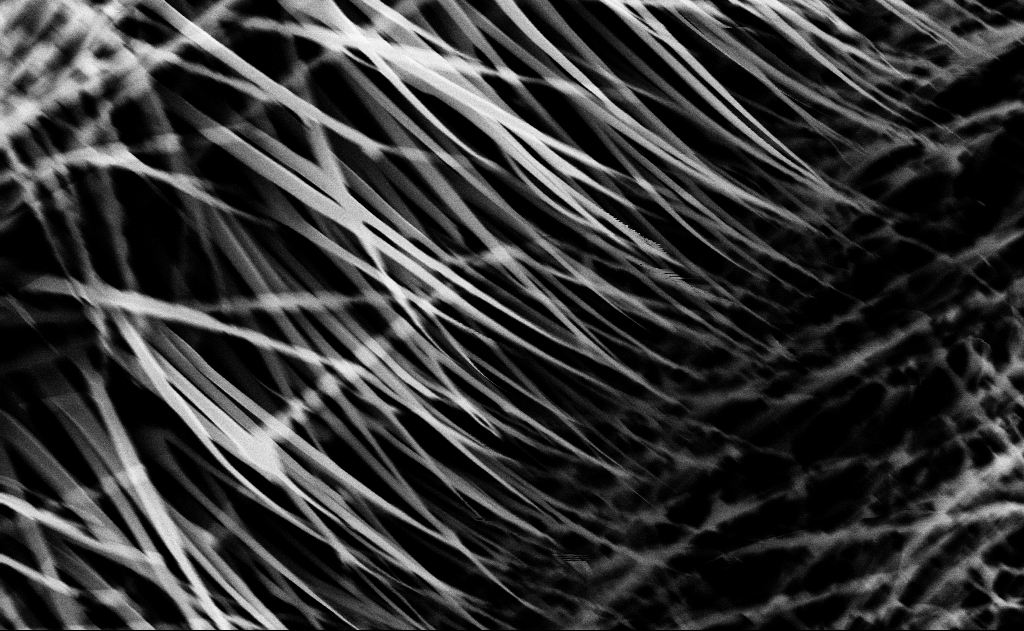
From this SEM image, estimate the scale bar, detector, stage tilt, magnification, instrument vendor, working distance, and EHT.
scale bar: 1000 nm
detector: InLens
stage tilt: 0°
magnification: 40 K X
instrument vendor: Zeiss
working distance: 13 mm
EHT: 10 kV